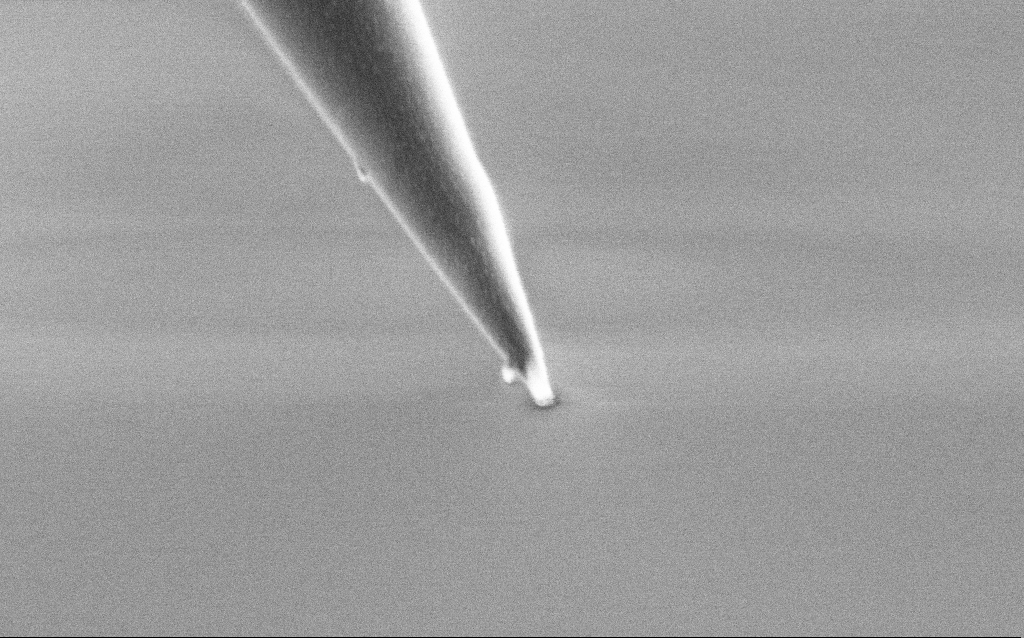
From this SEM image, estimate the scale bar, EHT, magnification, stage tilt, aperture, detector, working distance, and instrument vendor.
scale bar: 200 nm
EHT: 1 kV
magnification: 100 K X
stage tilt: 45°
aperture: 30 µm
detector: SE2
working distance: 7 mm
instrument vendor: Zeiss